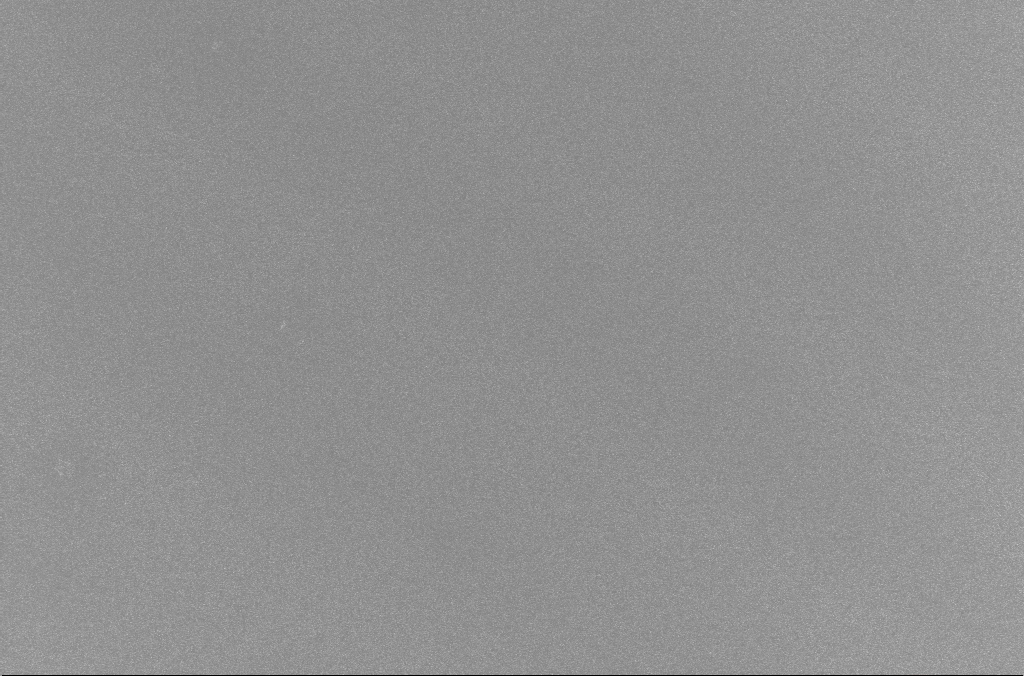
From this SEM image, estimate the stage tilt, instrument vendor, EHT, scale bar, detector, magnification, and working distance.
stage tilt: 0°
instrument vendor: Zeiss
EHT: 5 kV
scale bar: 20000 nm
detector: InLens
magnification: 1 K X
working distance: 3 mm